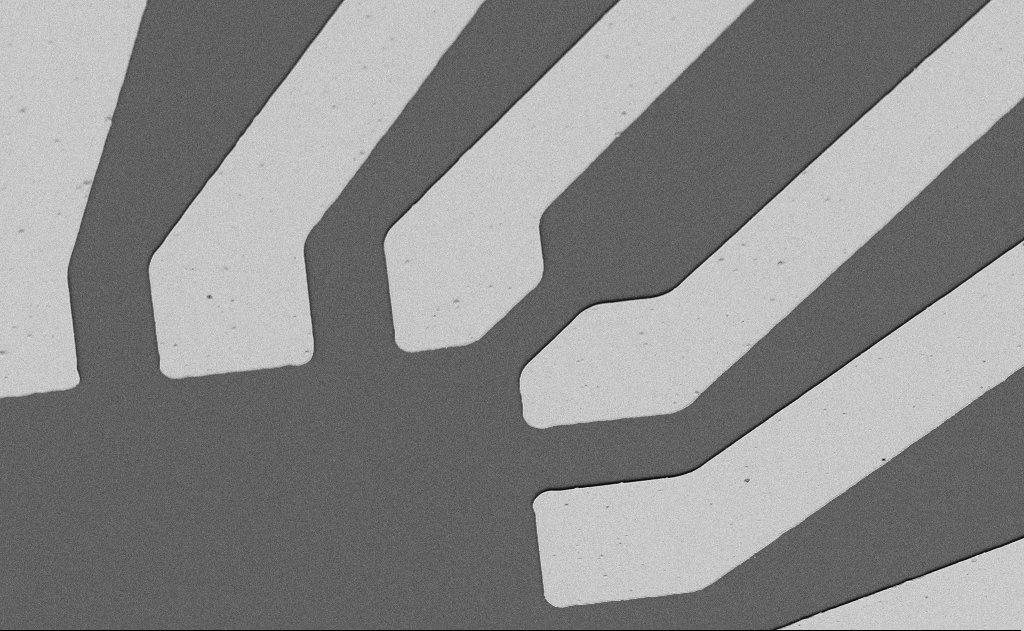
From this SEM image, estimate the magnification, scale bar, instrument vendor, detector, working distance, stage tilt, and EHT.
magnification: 2.9 K X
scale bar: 20000 nm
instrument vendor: Zeiss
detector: SE2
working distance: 8 mm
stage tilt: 39.8°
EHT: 5 kV